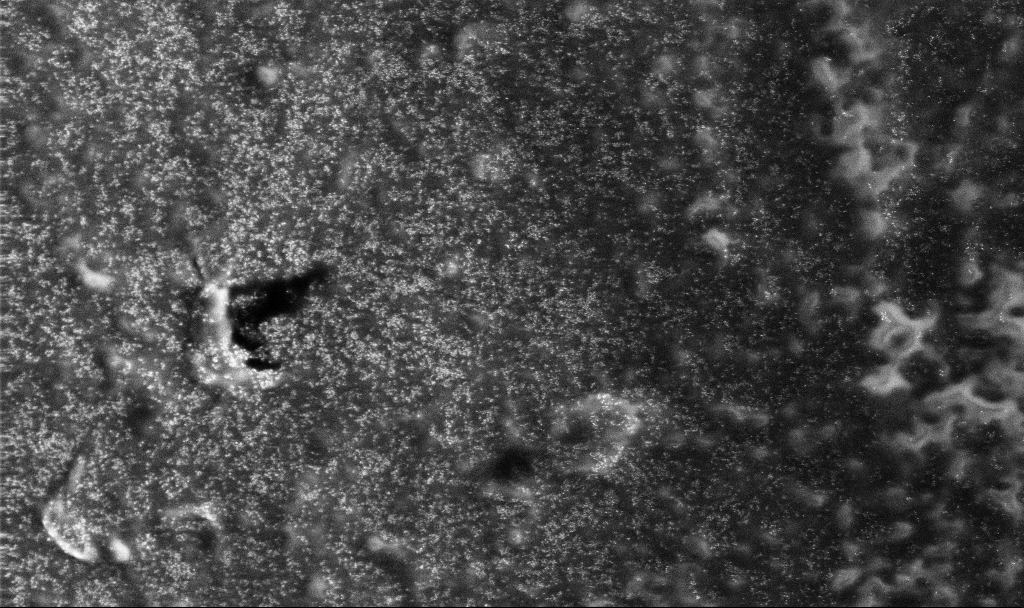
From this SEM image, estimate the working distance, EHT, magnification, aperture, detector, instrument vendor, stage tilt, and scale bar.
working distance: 2.4 mm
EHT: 10 kV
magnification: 1.83 K X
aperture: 30 µm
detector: InLens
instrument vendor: Zeiss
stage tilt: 0°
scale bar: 20000 nm